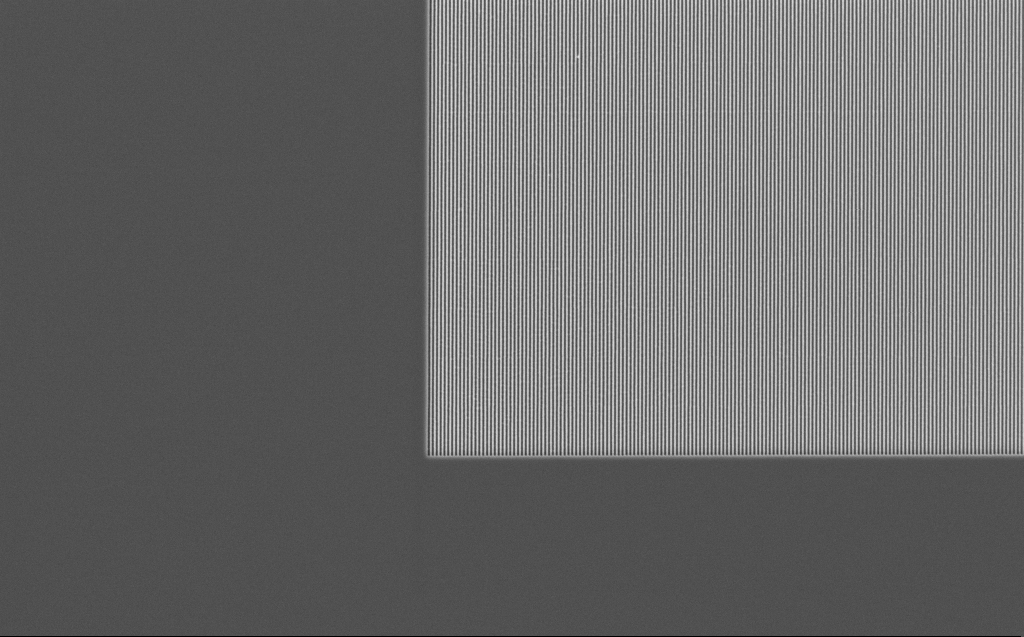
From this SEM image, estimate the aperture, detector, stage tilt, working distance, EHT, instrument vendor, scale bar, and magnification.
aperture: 30 µm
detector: InLens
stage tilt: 0°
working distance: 7 mm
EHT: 5 kV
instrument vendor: Zeiss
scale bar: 10000 nm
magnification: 7.13 K X